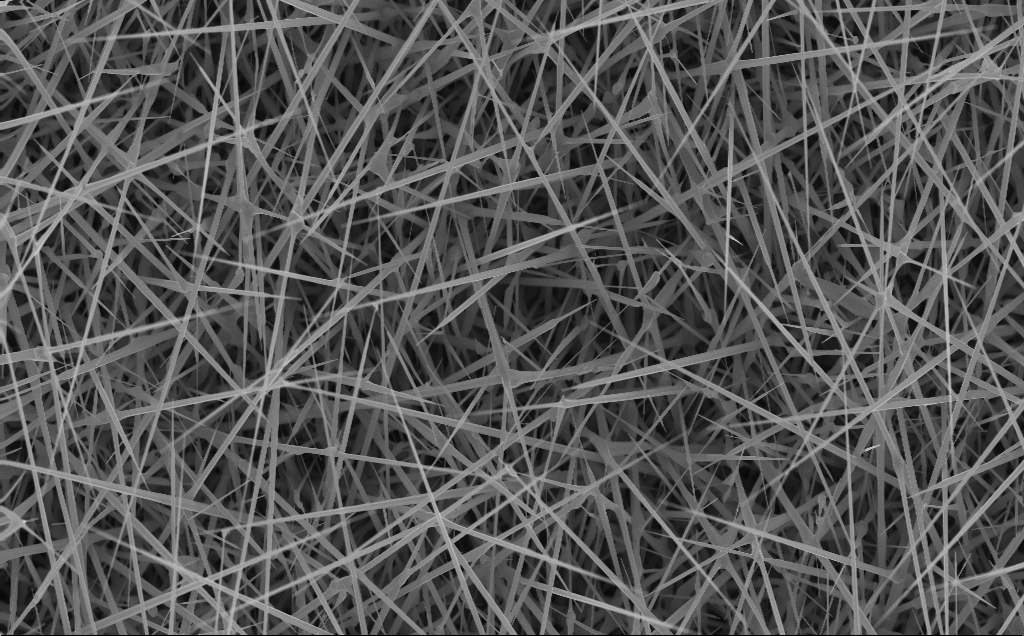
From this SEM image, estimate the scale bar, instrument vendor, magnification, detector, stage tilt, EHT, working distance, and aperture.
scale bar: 2000 nm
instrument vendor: Zeiss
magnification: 10 K X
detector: InLens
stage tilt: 0°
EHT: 10 kV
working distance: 4 mm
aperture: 30 µm